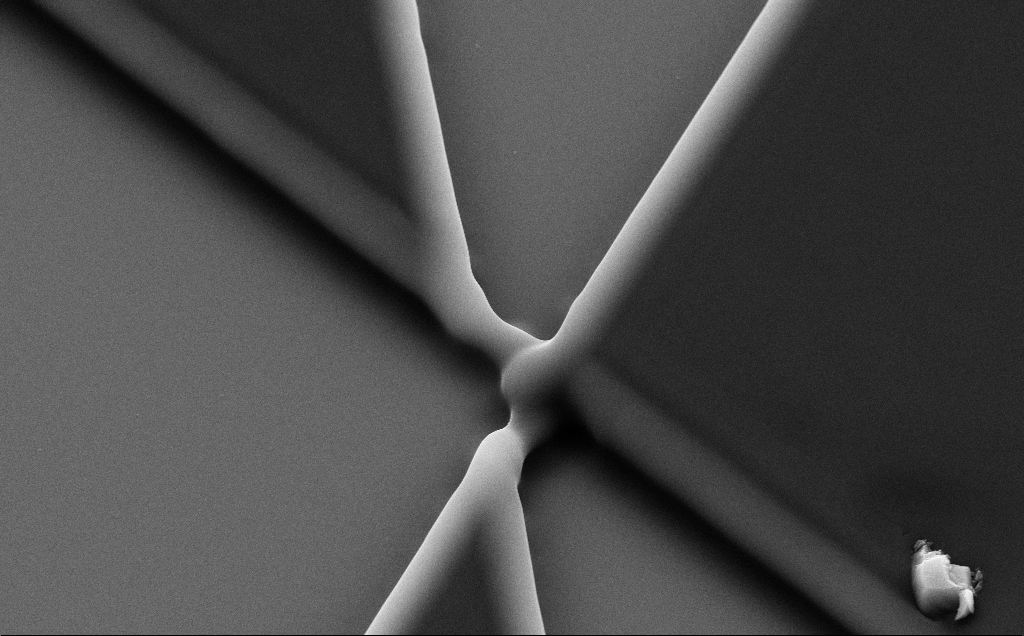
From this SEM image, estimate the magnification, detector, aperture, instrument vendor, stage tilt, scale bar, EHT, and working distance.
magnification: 12.36 K X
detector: SE2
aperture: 30 µm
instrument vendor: Zeiss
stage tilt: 35°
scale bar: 2000 nm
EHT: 10 kV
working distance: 8 mm